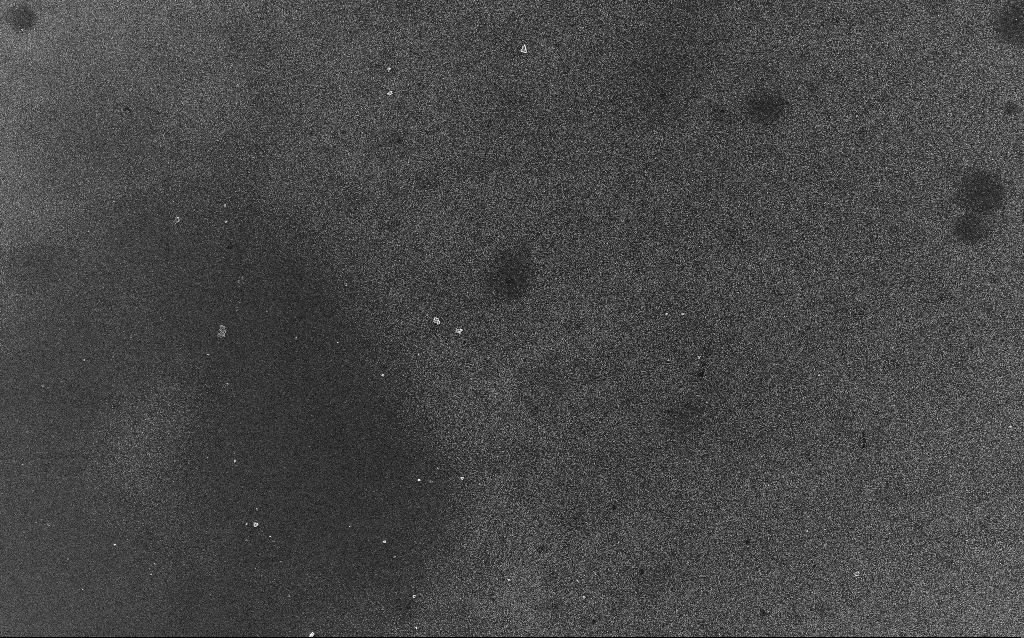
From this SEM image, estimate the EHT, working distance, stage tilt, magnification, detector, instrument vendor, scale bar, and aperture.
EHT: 5 kV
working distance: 2.1 mm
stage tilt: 0°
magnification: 1.22 K X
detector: InLens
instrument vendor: Zeiss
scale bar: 20000 nm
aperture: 30 µm